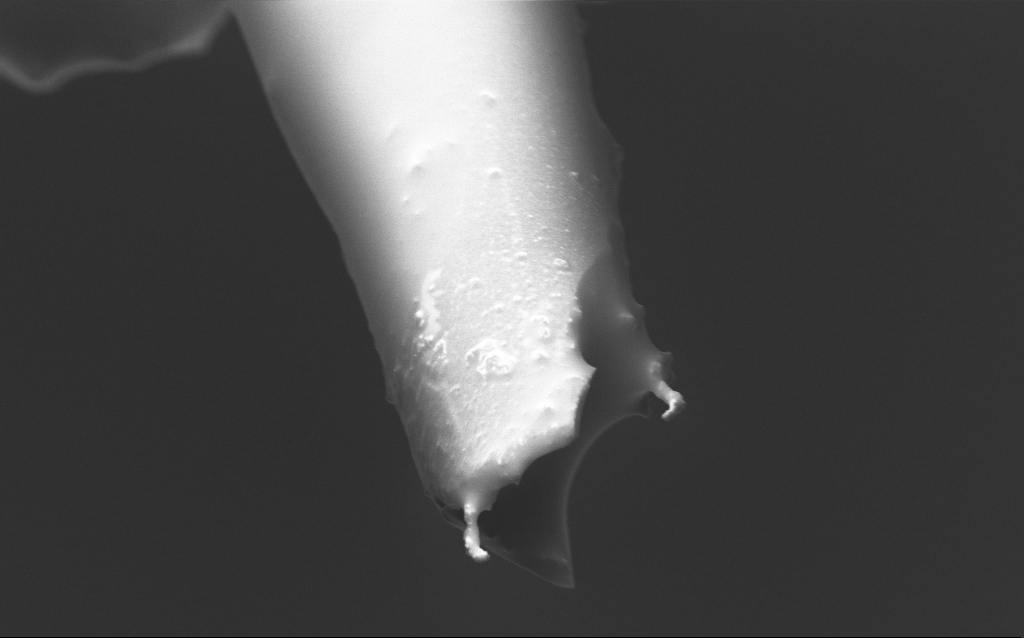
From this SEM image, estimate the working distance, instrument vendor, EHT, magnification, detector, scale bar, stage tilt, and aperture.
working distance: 5 mm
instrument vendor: Zeiss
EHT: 1.5 kV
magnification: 25 K X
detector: InLens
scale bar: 2000 nm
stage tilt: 45°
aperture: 30 µm